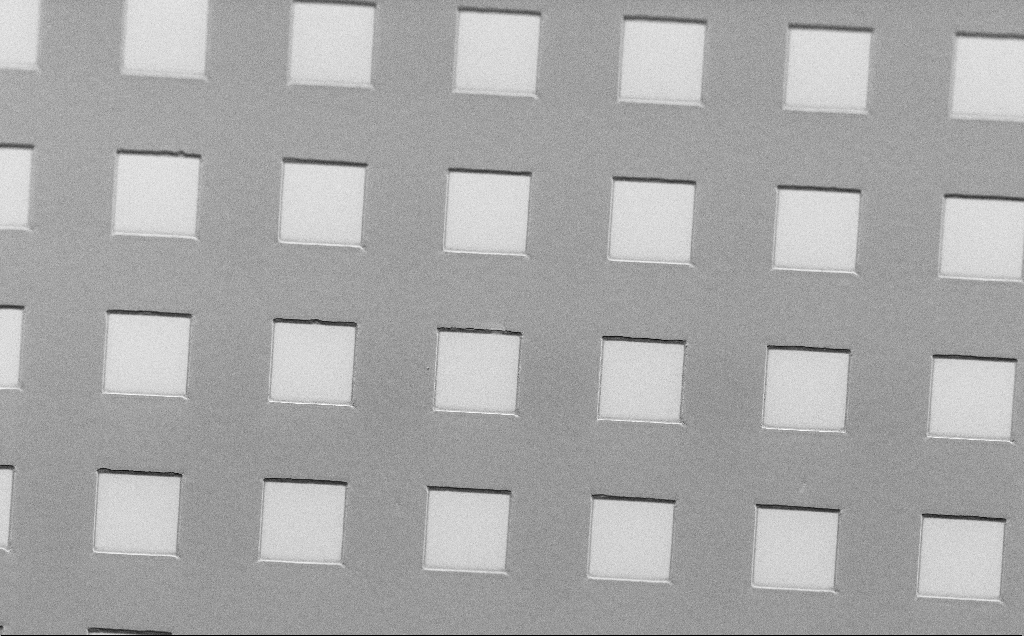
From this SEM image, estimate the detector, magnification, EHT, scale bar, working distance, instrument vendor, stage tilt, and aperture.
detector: SE2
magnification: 0.605 K X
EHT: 1.5 kV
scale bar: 100000 nm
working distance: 7 mm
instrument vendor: Zeiss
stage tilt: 45°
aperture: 30 µm